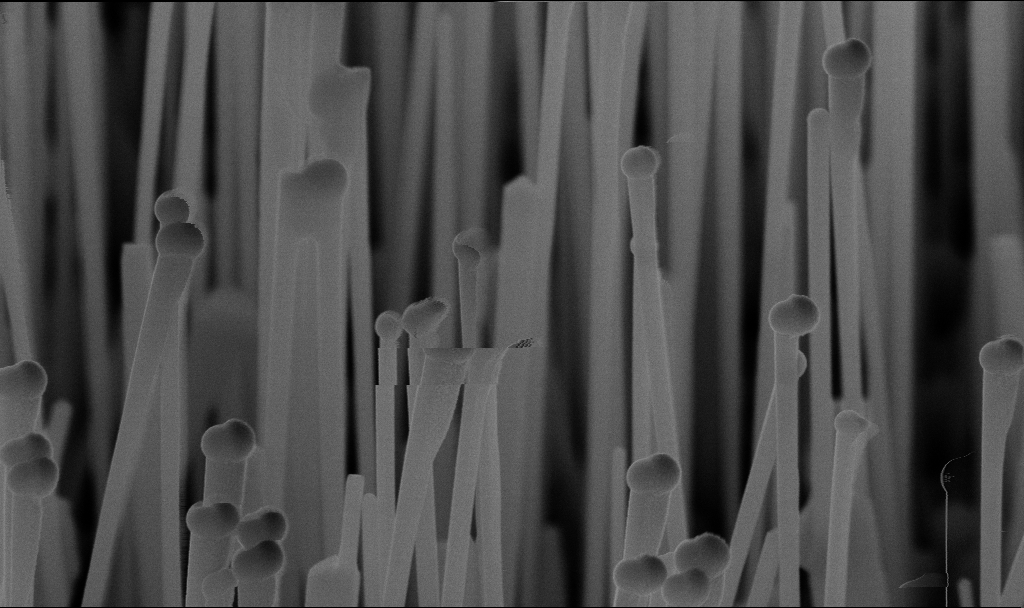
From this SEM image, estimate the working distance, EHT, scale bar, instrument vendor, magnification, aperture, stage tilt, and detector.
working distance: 7.1 mm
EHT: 10 kV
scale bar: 1000 nm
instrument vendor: Zeiss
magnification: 71.94 K X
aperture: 30 µm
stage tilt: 45°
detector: InLens